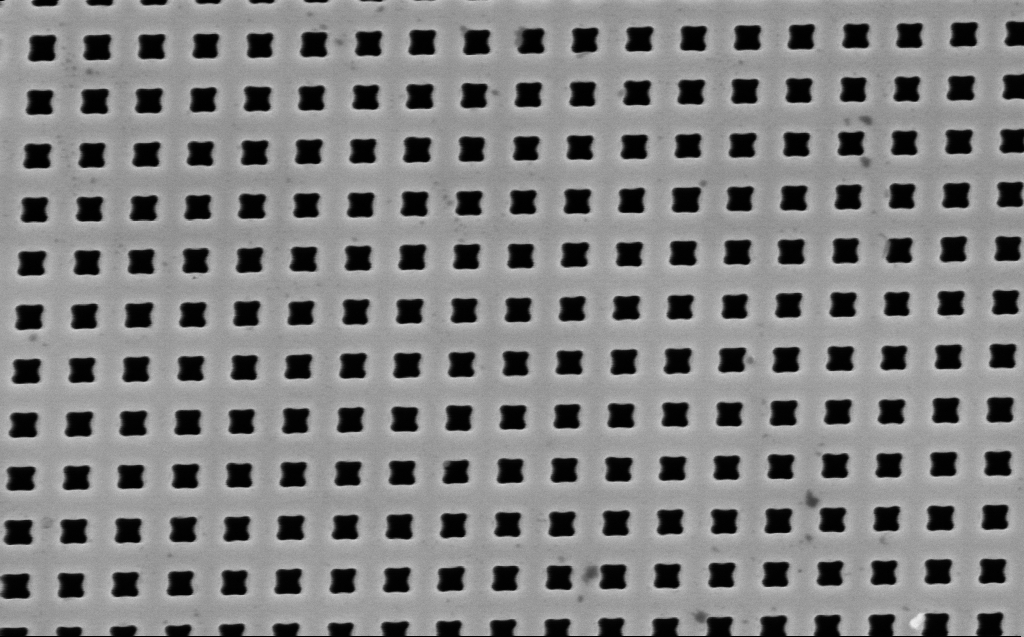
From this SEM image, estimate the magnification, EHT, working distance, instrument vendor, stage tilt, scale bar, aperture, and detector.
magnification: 40 K X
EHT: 3 kV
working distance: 6 mm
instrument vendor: Zeiss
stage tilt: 0°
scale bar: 1000 nm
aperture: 30 µm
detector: InLens